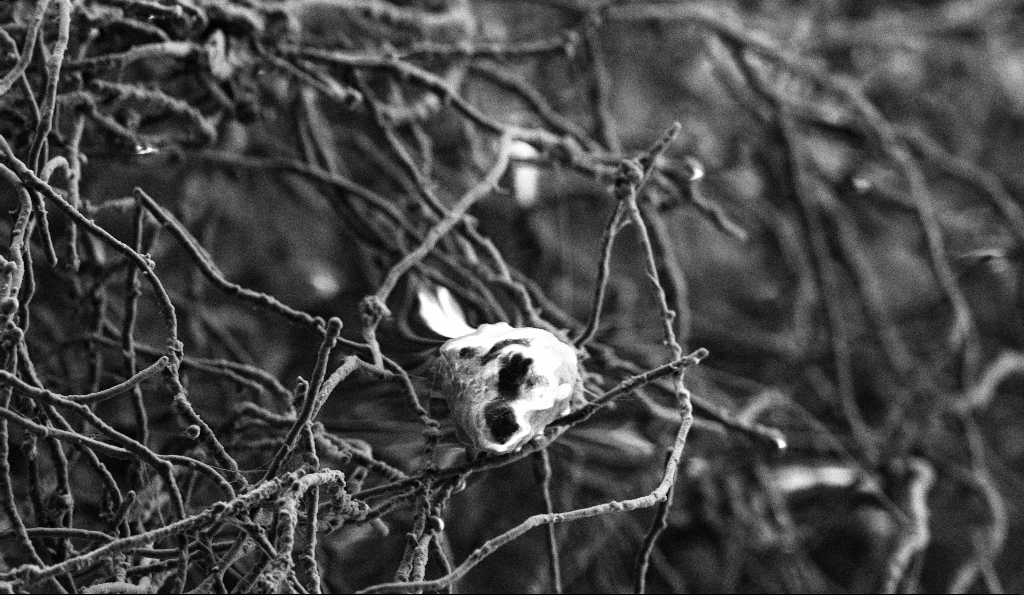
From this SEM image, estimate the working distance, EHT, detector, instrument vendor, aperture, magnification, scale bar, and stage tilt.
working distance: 4.8 mm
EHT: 3 kV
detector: SE2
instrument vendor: Zeiss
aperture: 30 µm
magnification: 5 K X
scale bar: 10000 nm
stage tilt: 0°